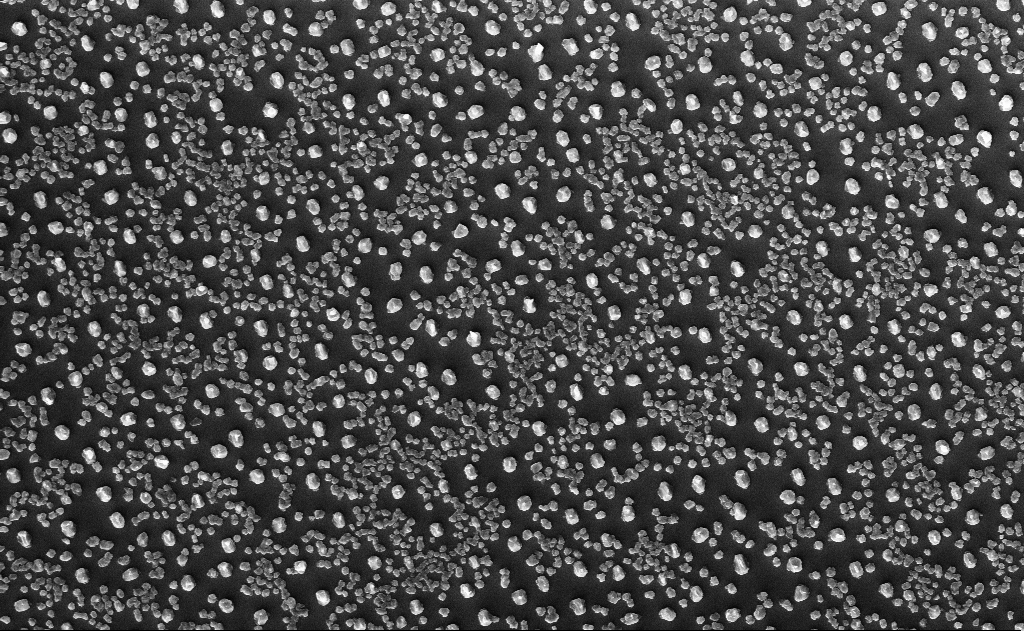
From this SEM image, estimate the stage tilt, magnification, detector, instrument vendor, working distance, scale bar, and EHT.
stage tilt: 0°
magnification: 10 K X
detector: InLens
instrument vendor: Zeiss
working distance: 18 mm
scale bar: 2000 nm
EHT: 10 kV